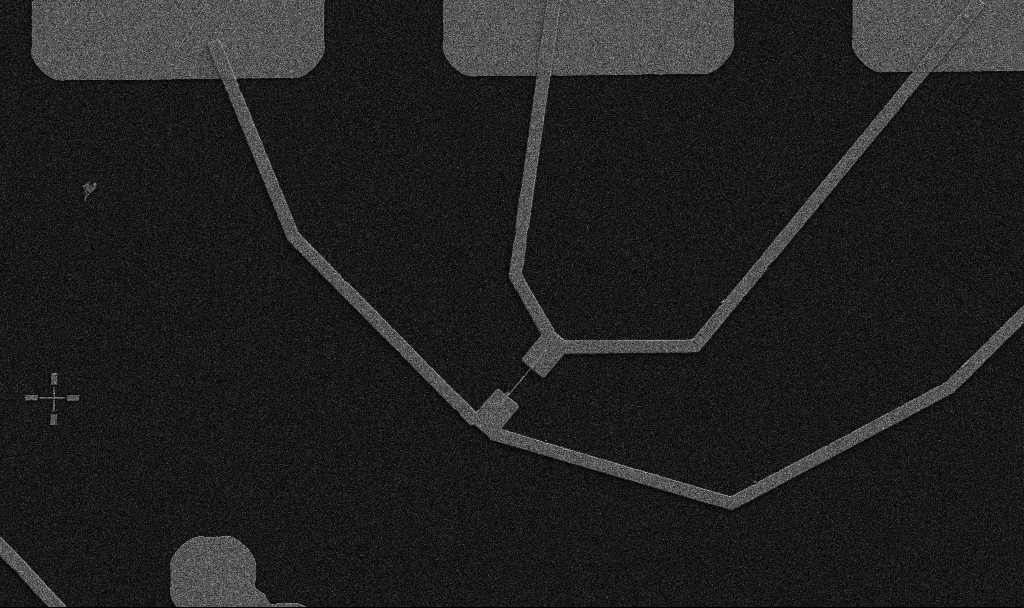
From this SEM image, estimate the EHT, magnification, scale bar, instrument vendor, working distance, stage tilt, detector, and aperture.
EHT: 5 kV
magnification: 5 K X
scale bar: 10000 nm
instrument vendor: Zeiss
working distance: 10.7 mm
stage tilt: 0°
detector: SE2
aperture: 30 µm